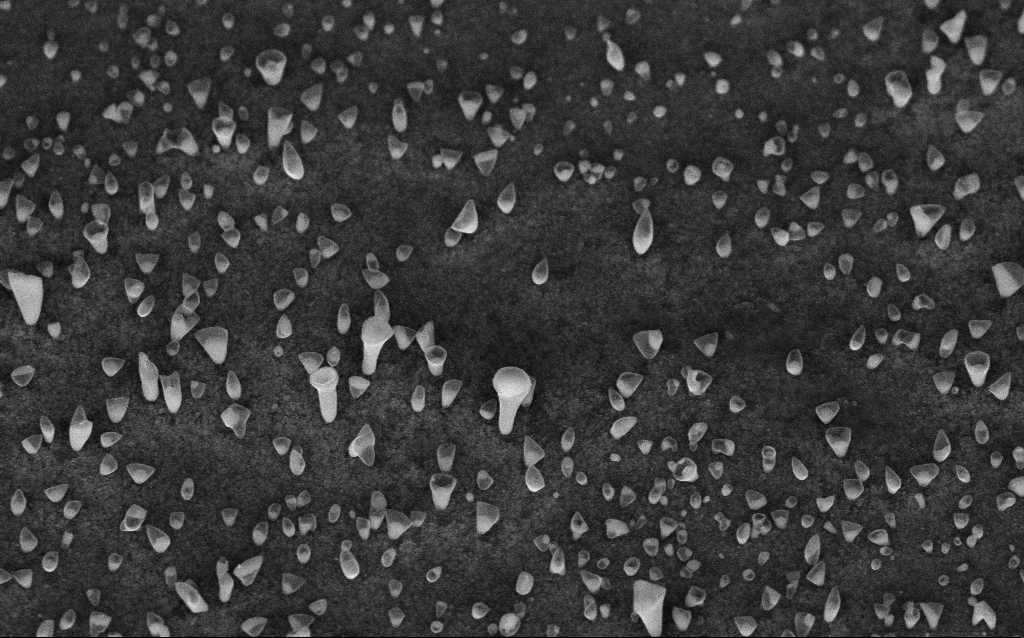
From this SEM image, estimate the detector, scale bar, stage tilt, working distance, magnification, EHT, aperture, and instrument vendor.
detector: InLens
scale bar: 1000 nm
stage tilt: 45°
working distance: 7.3 mm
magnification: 50 K X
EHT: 5 kV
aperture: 30 µm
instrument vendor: Zeiss